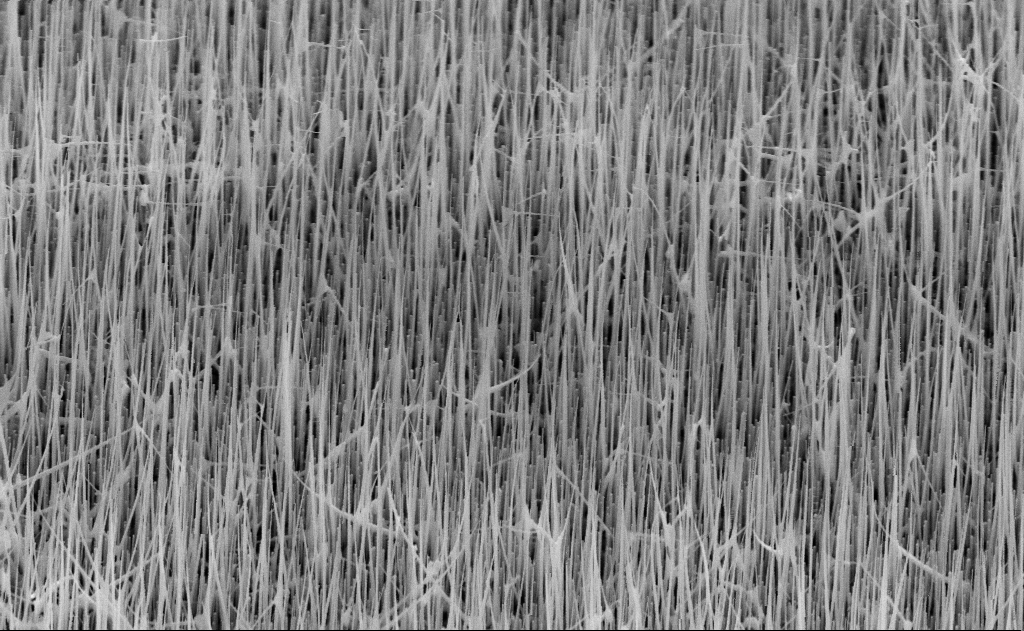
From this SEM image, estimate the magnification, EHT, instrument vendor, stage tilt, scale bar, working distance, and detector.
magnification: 20 K X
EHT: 10 kV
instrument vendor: Zeiss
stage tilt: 45°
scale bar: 1000 nm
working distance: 16 mm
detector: SE2